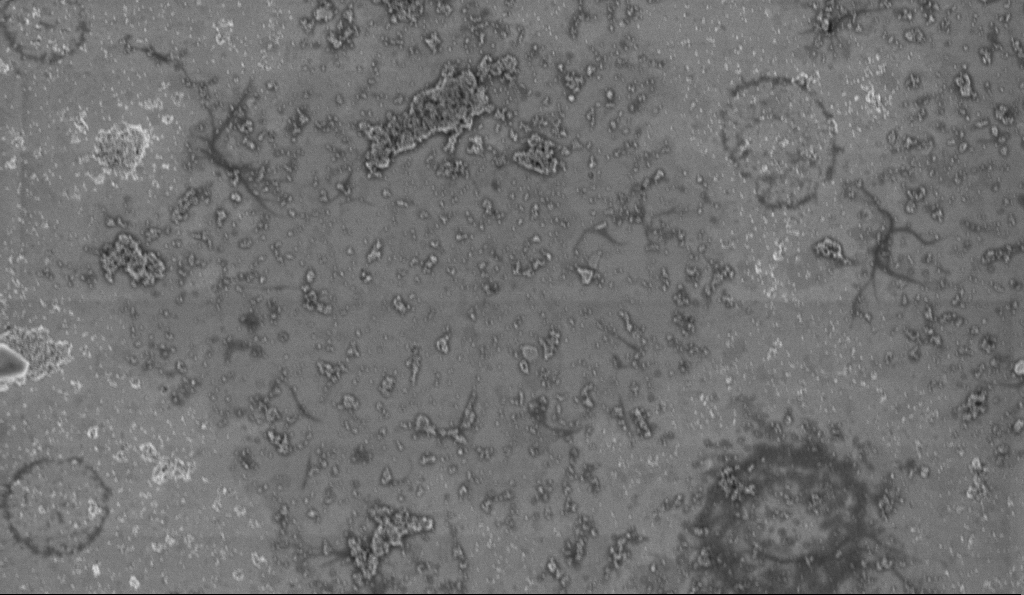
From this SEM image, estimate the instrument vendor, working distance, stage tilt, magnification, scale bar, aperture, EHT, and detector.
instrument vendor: Zeiss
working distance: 3.2 mm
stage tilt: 0°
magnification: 25 K X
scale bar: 1000 nm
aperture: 30 µm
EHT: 2 kV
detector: InLens